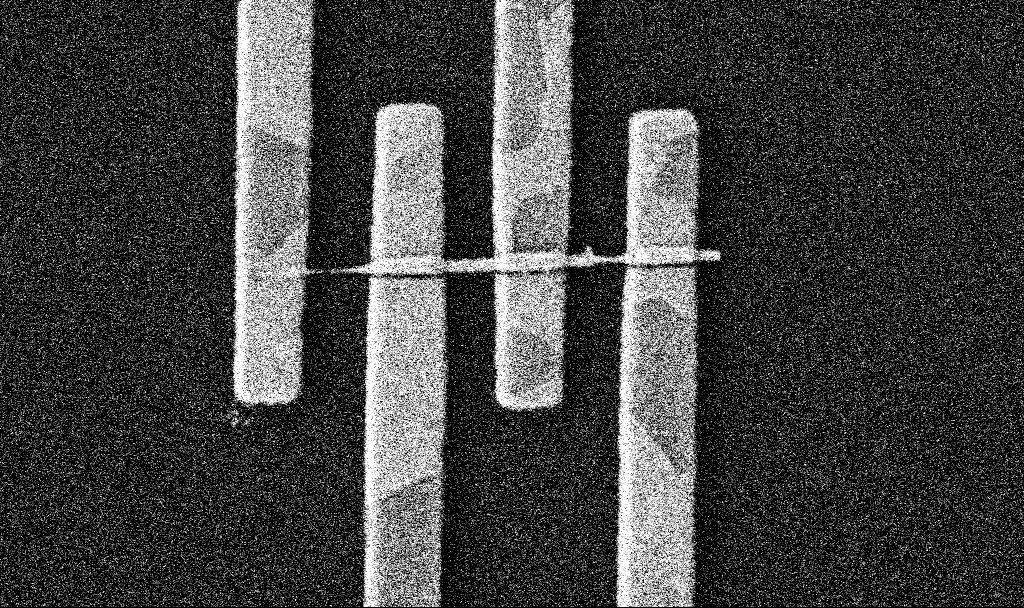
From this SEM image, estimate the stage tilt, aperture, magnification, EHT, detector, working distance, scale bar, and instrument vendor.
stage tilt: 0°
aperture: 30 µm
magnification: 42.97 K X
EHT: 5 kV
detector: SE2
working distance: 8.5 mm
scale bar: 1000 nm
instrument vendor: Zeiss